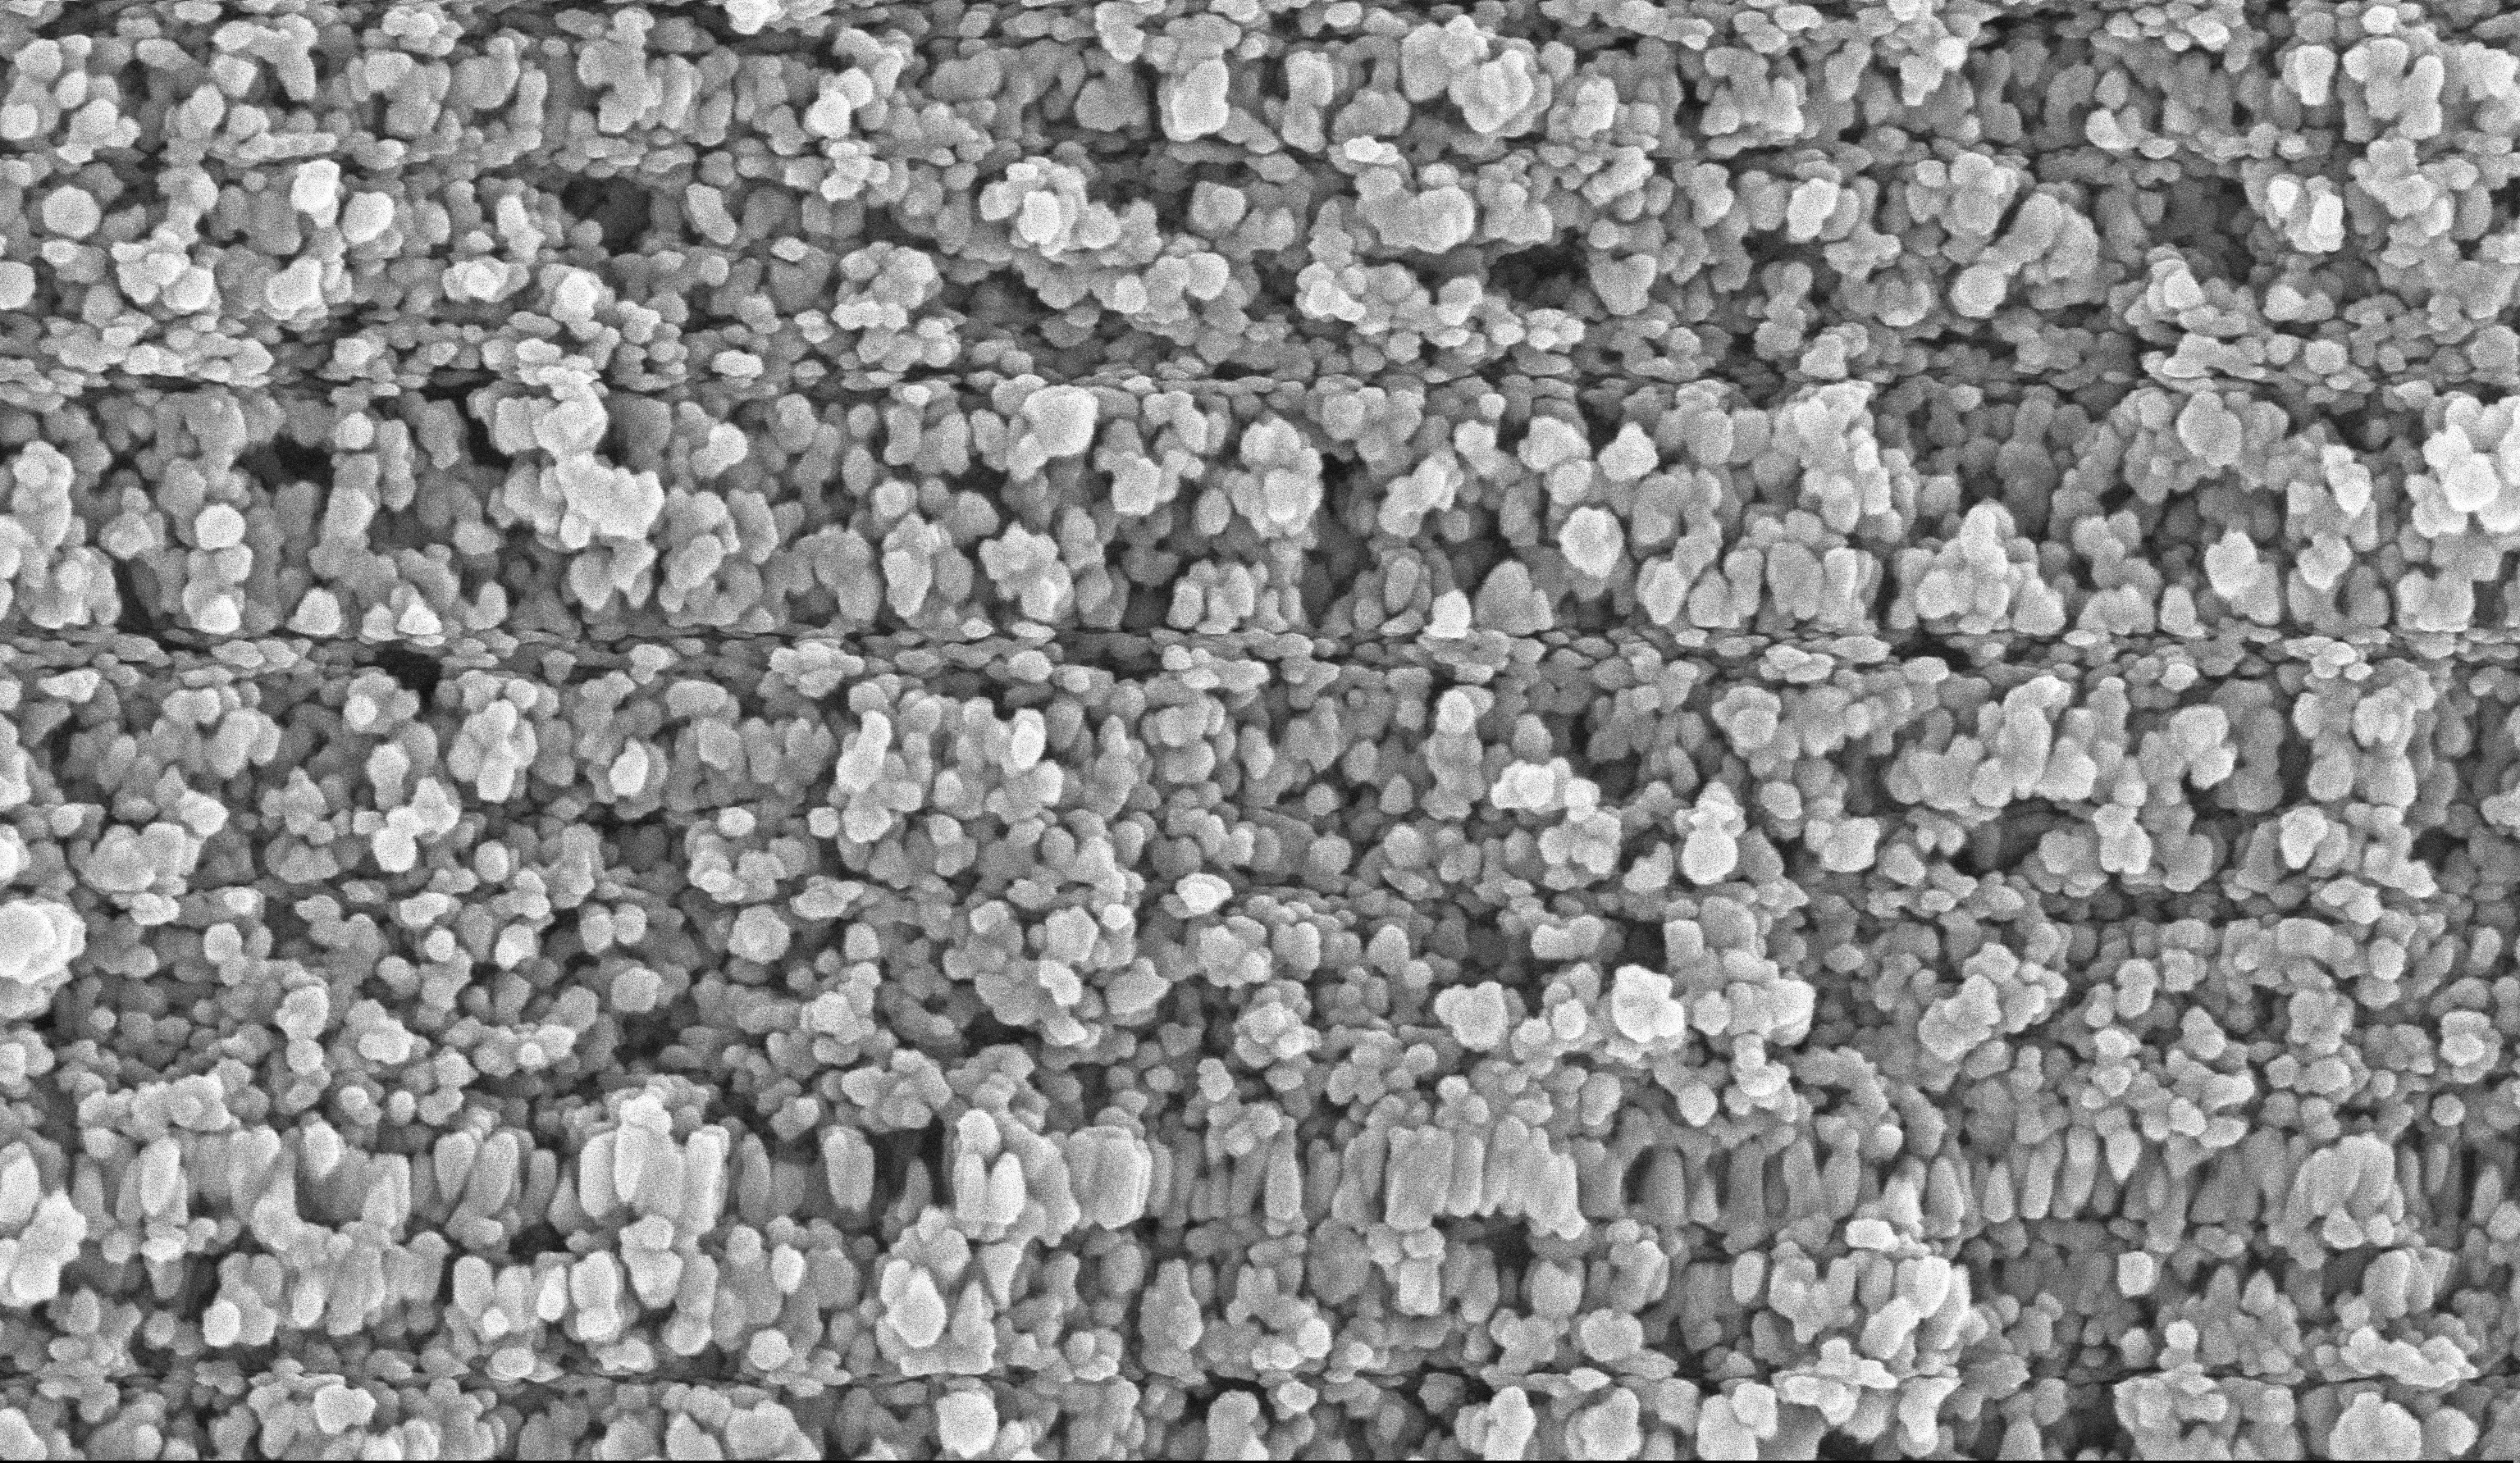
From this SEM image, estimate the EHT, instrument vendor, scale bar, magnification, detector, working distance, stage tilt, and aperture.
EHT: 10 kV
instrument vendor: Zeiss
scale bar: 200 nm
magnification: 135 K X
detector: InLens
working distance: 6 mm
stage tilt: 0°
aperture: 30 µm